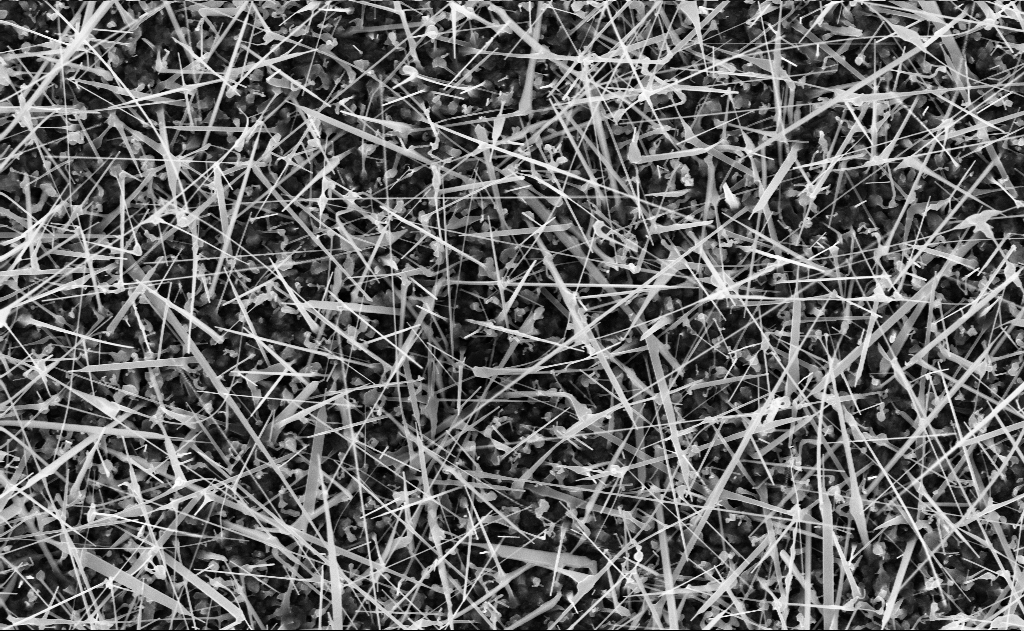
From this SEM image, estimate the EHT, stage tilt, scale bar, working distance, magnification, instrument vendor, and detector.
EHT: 10 kV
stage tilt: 0°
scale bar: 1000 nm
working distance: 11 mm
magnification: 20 K X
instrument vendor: Zeiss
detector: InLens